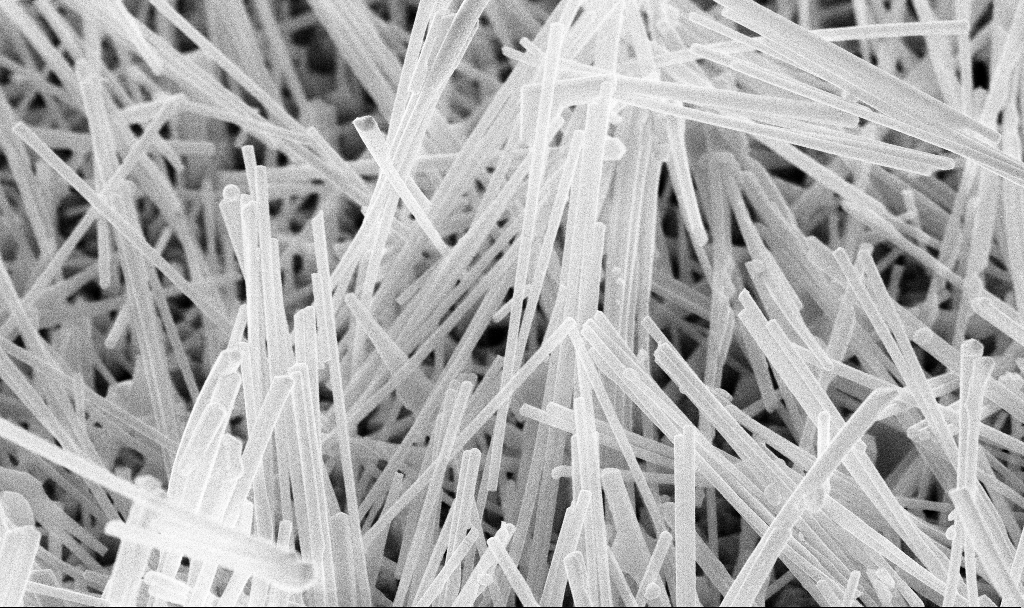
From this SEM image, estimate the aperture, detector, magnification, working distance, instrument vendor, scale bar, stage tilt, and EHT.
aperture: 30 µm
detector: InLens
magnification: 35.36 K X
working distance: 7 mm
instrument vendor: Zeiss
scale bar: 2000 nm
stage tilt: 45°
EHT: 10 kV